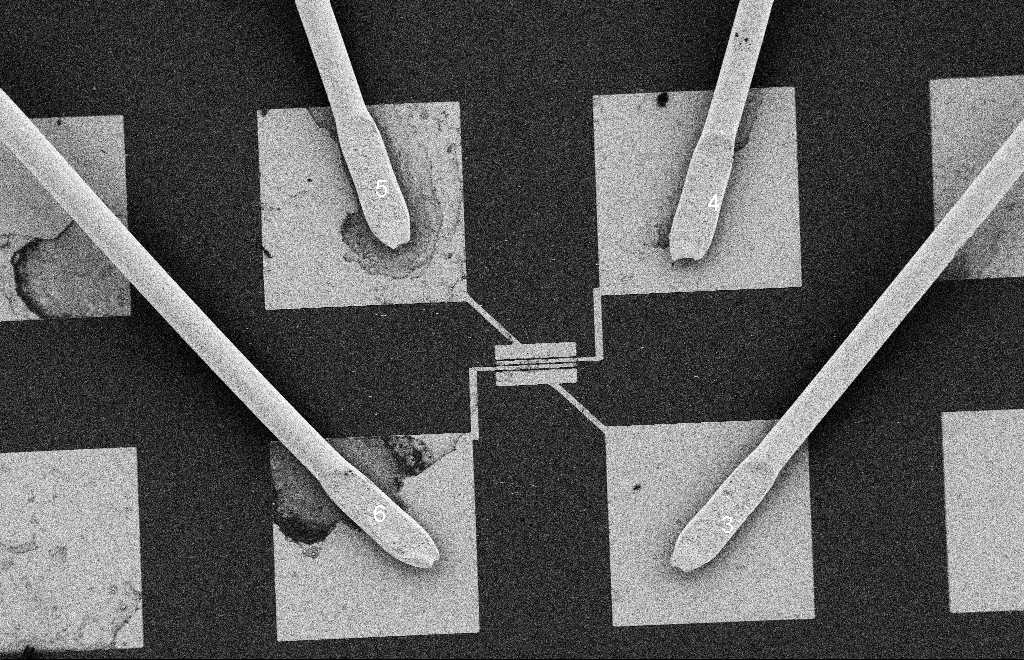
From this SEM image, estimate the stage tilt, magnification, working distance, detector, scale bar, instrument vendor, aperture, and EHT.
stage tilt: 0°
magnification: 0.486 K X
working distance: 9 mm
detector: SE2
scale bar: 20000 nm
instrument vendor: Zeiss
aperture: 20 µm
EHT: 2 kV